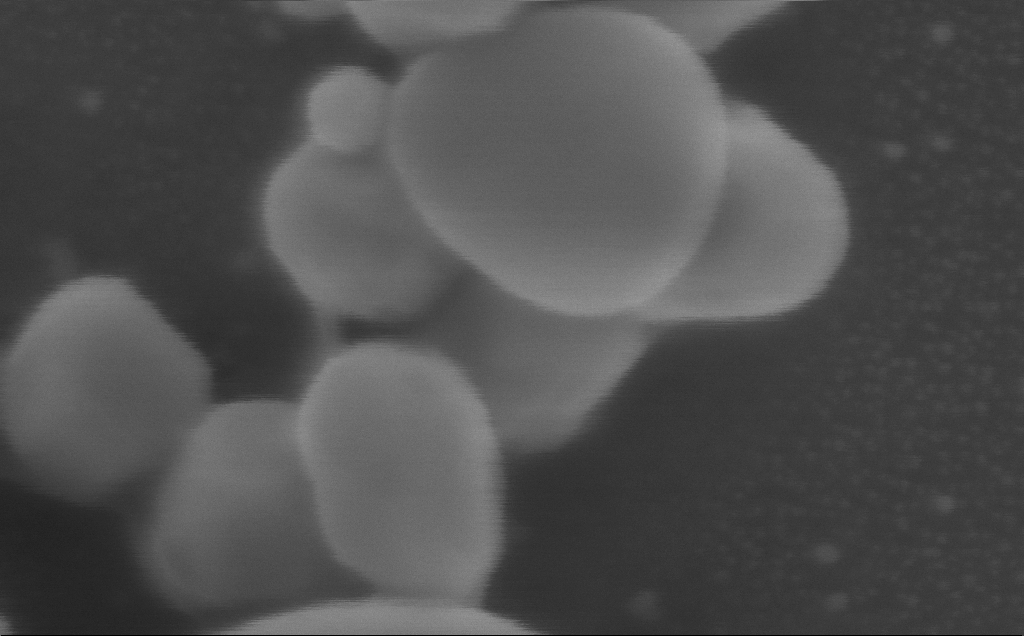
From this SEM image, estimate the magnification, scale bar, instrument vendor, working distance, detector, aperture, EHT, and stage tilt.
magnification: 600 K X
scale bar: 100 nm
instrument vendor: Zeiss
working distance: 3 mm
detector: InLens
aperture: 30 µm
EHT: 5 kV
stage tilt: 0°